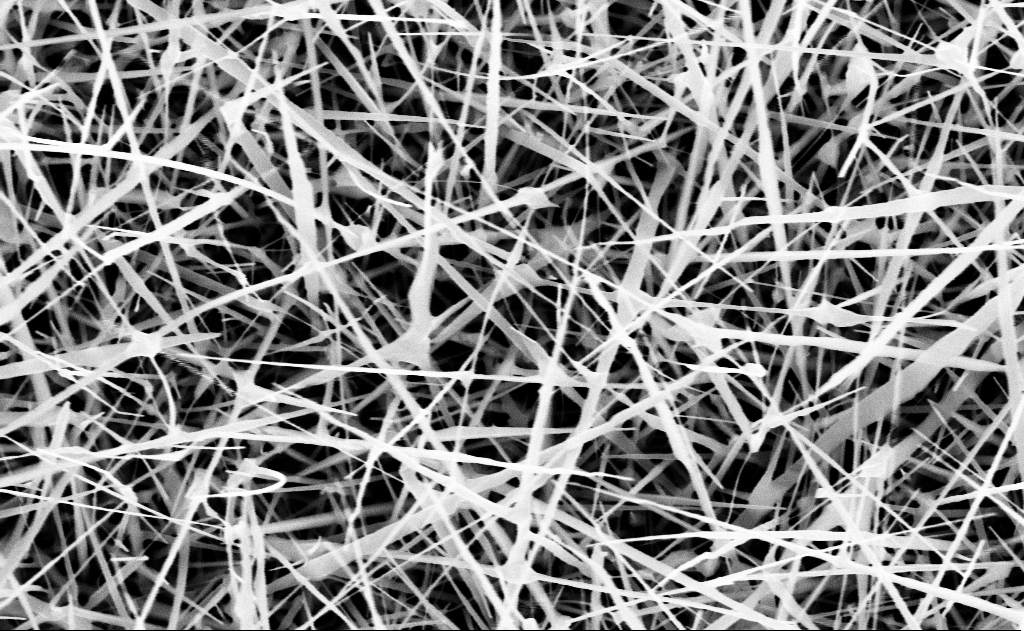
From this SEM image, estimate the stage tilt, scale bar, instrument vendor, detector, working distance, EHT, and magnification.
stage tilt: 0°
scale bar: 1000 nm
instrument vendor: Zeiss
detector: InLens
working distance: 15 mm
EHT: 10 kV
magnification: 40 K X